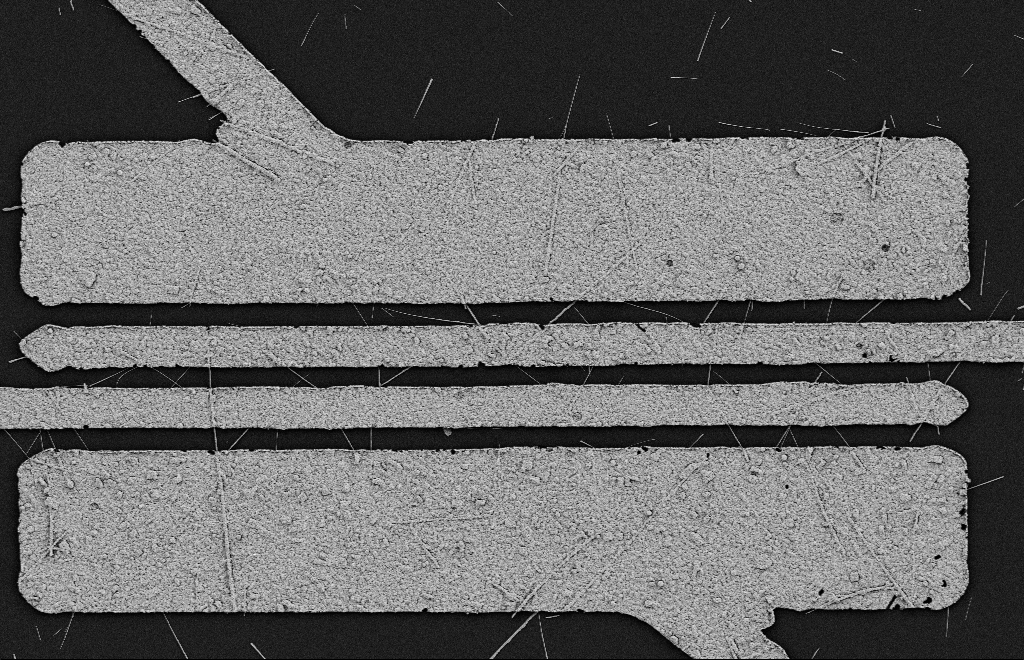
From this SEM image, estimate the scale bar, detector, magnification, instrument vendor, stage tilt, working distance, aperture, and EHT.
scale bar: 2000 nm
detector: SE2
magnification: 5.66 K X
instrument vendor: Zeiss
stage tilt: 0°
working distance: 11 mm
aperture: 20 µm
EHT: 2 kV